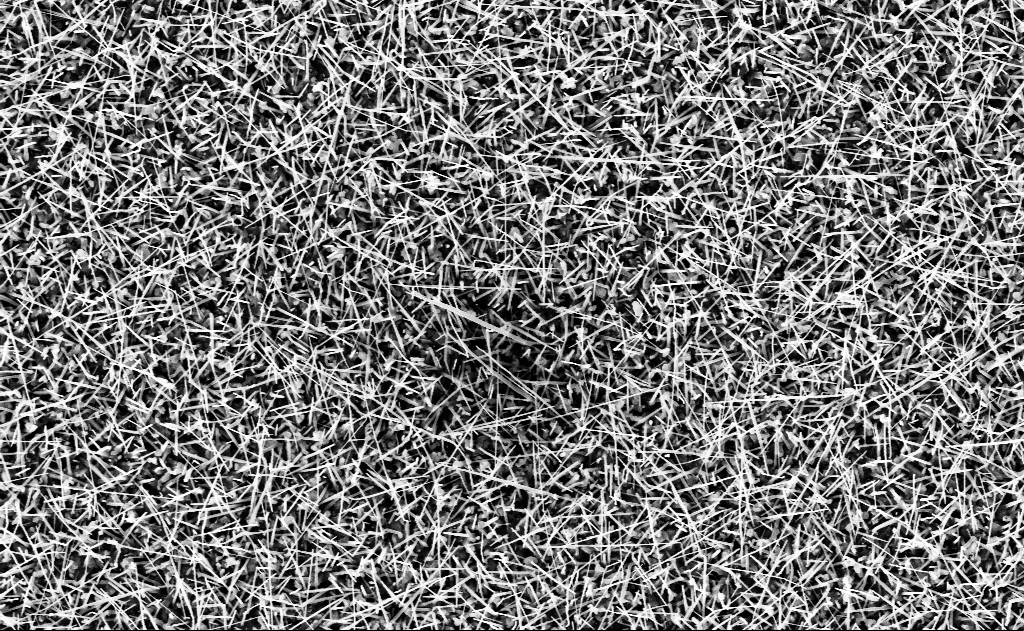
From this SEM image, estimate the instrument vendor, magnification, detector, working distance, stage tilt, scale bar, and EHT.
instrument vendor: Zeiss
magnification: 20 K X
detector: InLens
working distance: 15 mm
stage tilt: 0°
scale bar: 1000 nm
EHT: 10 kV